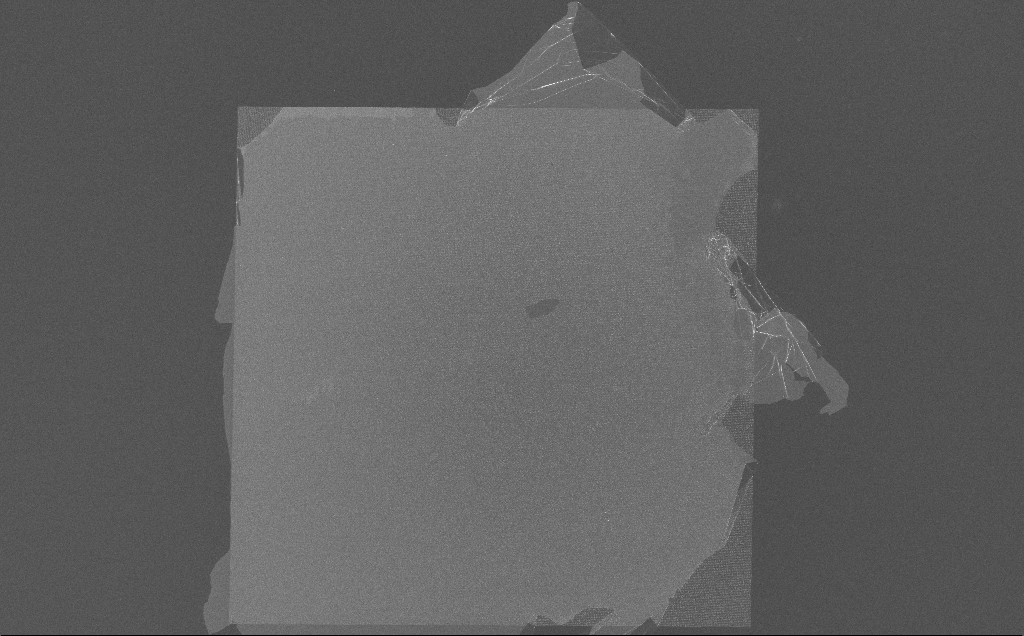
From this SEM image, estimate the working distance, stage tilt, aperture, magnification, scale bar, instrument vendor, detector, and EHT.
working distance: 7 mm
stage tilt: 0°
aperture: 30 µm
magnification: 0.257 K X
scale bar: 100000 nm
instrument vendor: Zeiss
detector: InLens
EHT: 10 kV